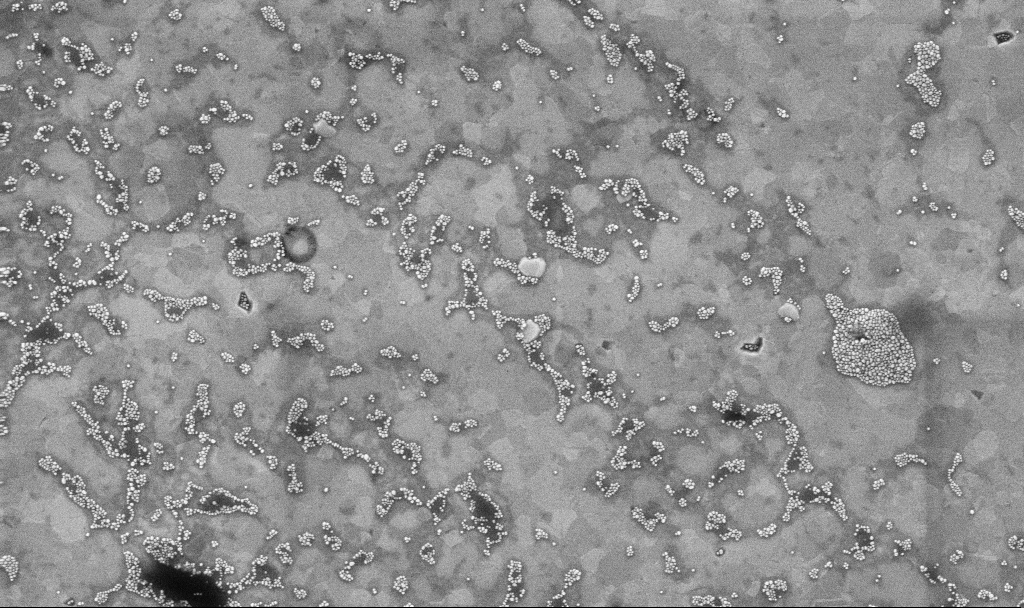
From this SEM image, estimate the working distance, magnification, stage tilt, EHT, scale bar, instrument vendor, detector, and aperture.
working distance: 3.1 mm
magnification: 50.18 K X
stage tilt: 0°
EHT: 10 kV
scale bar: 1000 nm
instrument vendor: Zeiss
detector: InLens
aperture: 30 µm